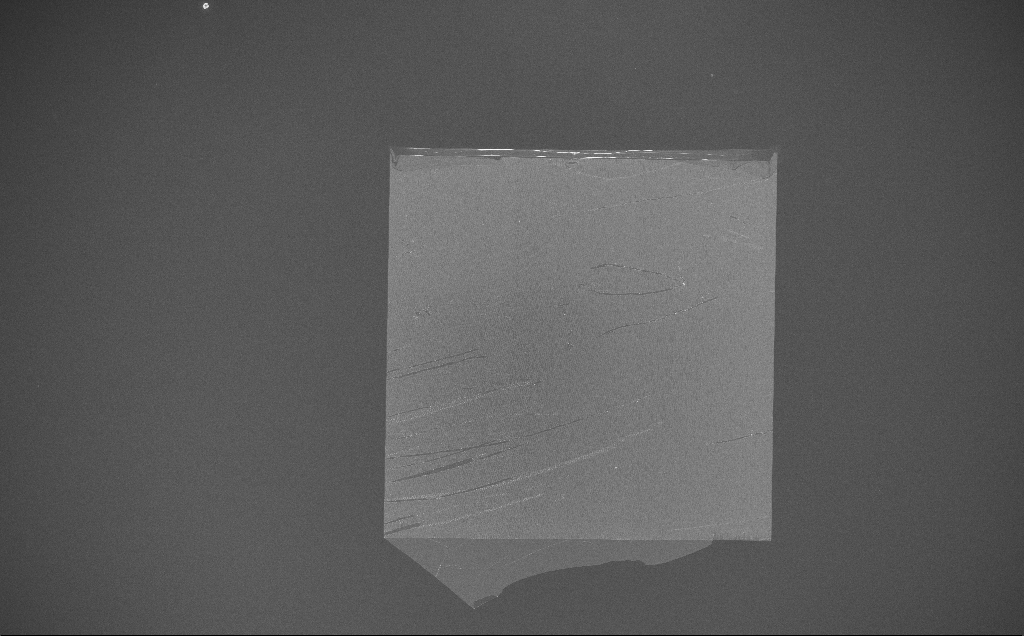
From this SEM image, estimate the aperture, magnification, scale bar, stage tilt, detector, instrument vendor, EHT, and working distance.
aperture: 30 µm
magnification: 0.147 K X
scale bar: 100000 nm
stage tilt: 0°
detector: InLens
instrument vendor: Zeiss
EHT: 10 kV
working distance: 7 mm